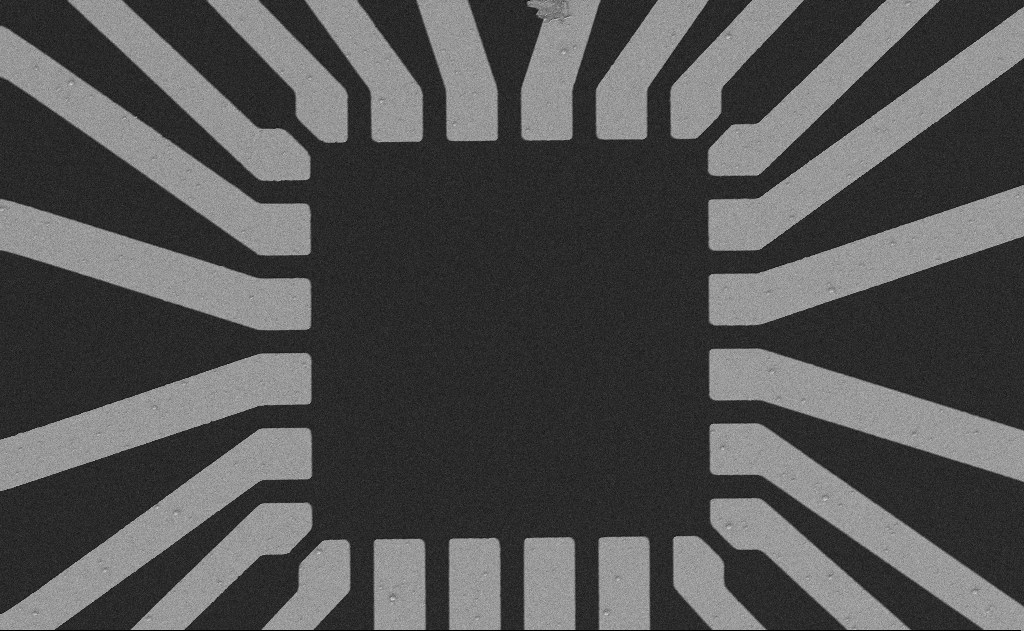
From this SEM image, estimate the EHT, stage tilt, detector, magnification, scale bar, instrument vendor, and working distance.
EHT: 5 kV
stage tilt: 0°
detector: SE2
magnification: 0.921 K X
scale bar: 20000 nm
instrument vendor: Zeiss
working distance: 7 mm